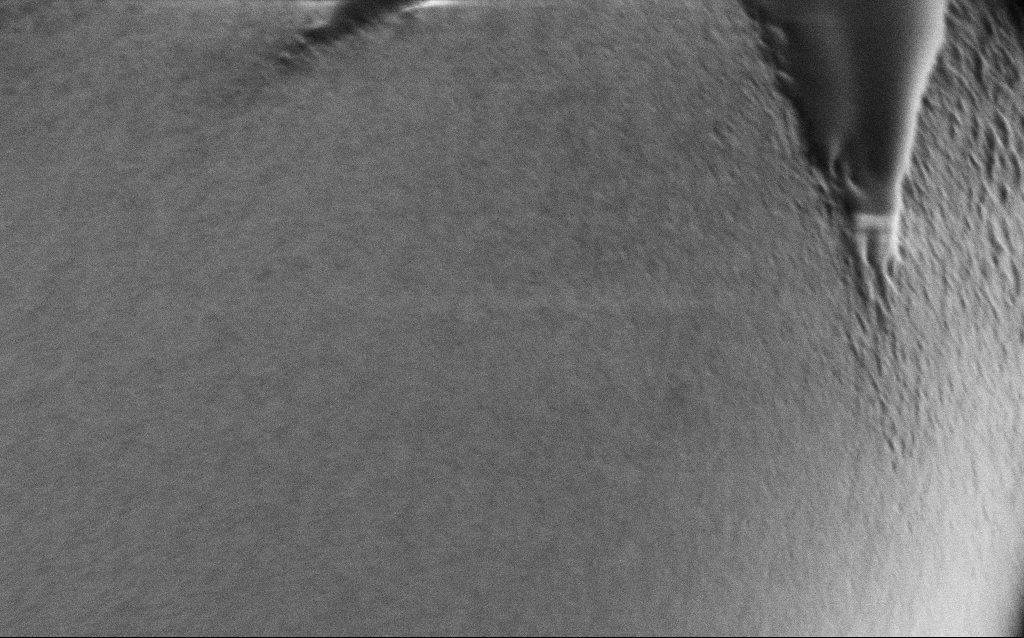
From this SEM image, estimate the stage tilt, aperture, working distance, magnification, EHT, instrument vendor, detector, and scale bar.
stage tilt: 45°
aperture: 30 µm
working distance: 6.1 mm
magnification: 40 K X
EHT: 1 kV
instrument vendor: Zeiss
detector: SE2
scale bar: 1000 nm